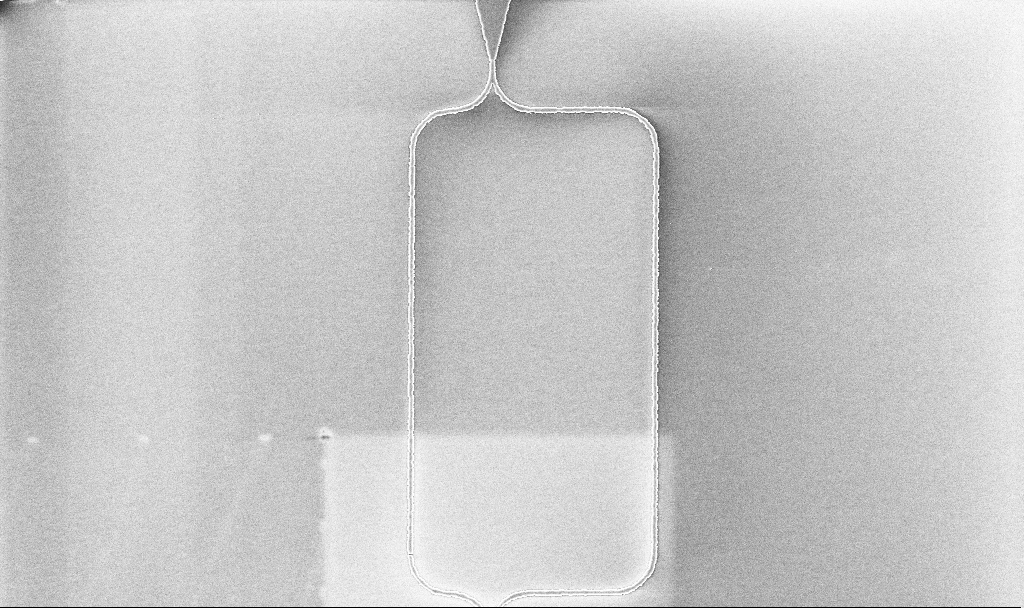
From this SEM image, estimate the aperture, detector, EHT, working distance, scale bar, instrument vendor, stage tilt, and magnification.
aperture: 30 µm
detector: InLens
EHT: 5 kV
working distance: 10.1 mm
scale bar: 10000 nm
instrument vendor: Zeiss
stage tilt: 0°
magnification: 3.11 K X